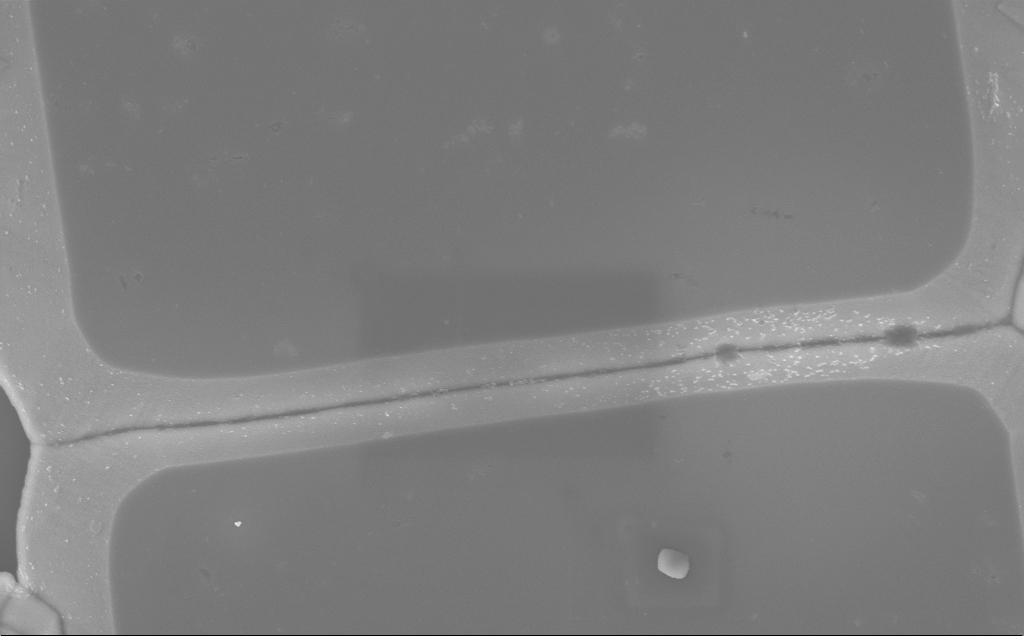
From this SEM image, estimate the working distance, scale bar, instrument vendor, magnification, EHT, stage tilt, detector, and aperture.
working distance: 10 mm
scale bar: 2000 nm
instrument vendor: Zeiss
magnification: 16.61 K X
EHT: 5 kV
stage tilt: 0°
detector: InLens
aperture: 30 µm